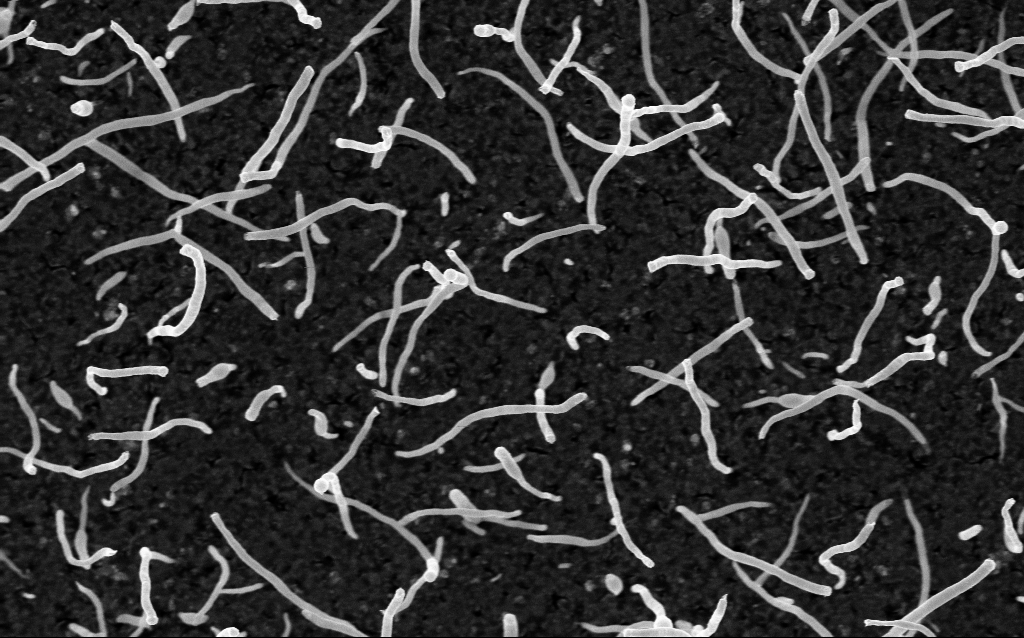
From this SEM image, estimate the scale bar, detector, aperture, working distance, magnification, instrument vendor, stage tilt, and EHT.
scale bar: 1000 nm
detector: InLens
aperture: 30 µm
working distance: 1.8 mm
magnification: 50 K X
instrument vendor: Zeiss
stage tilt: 0°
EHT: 5 kV